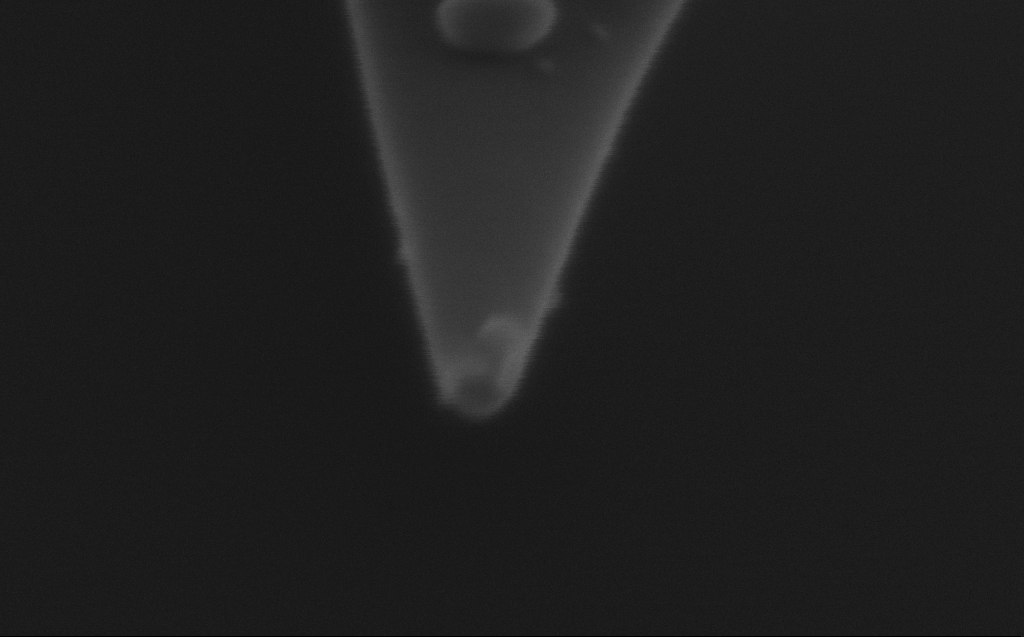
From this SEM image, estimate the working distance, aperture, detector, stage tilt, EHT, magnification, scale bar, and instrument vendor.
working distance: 3 mm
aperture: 20 µm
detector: InLens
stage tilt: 45.1°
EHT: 1.75 kV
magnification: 404.82 K X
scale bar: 100 nm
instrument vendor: Zeiss